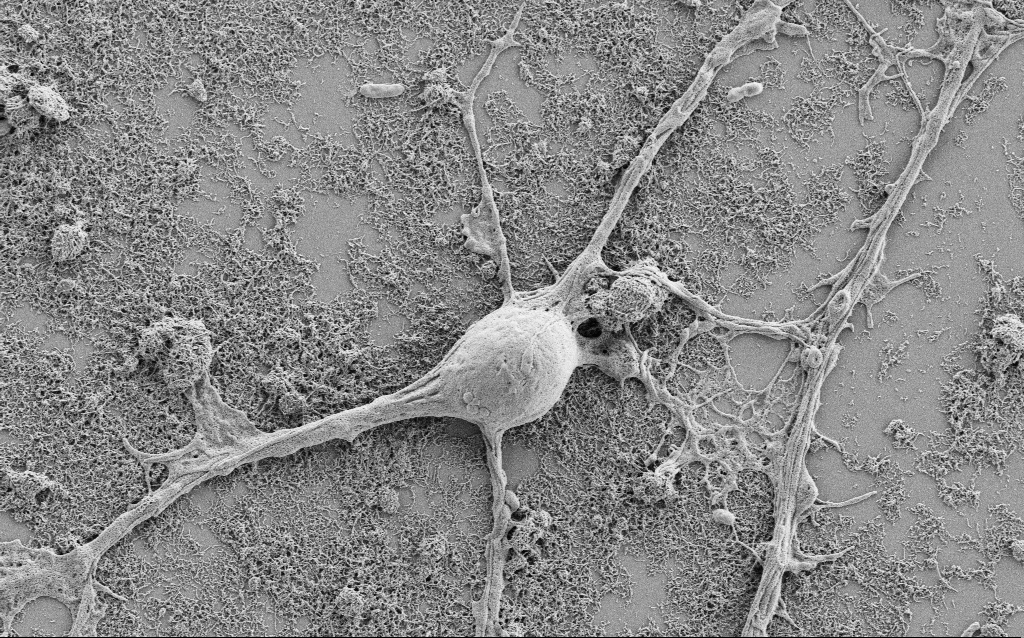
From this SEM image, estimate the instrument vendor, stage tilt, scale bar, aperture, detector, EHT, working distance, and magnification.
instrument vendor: Zeiss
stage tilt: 0°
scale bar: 2000 nm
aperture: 30 µm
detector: SE2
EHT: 1.5 kV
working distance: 6.9 mm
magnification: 7.5 K X